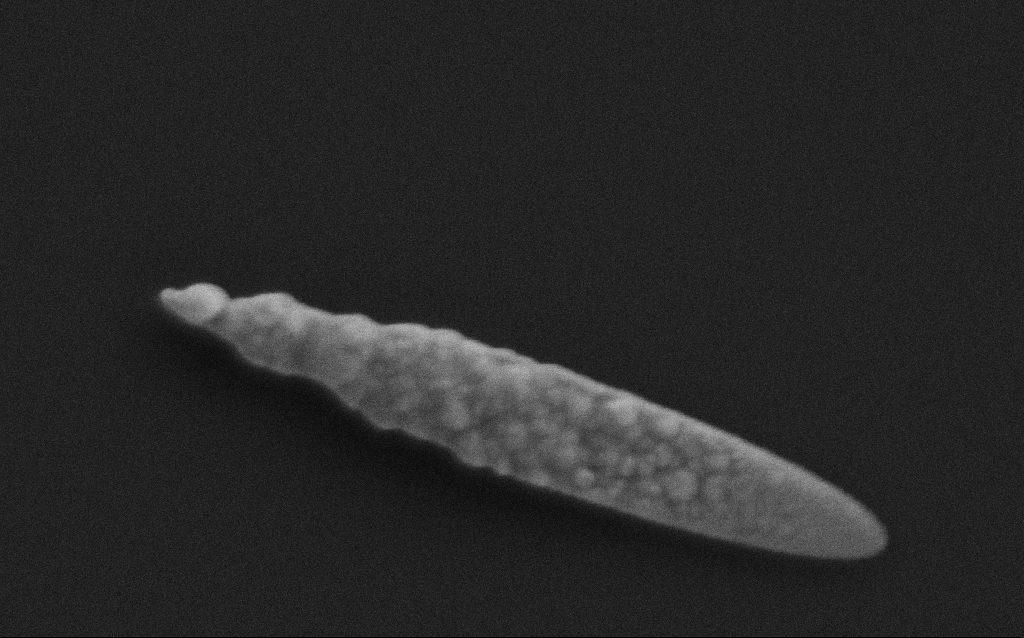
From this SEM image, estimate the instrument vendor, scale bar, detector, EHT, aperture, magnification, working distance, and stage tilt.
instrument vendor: Zeiss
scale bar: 200 nm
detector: SE2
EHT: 3 kV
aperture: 30 µm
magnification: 112.37 K X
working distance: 3 mm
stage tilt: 0°